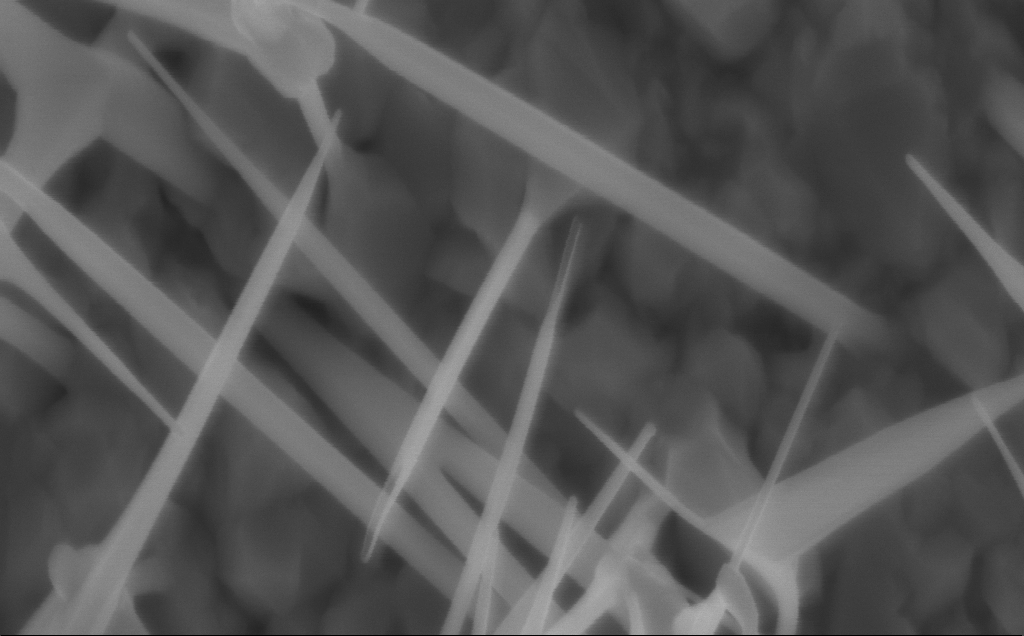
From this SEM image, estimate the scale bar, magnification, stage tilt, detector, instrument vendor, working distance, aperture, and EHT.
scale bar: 100 nm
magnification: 150 K X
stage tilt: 0°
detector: InLens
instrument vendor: Zeiss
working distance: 5 mm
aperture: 30 µm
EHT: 5 kV